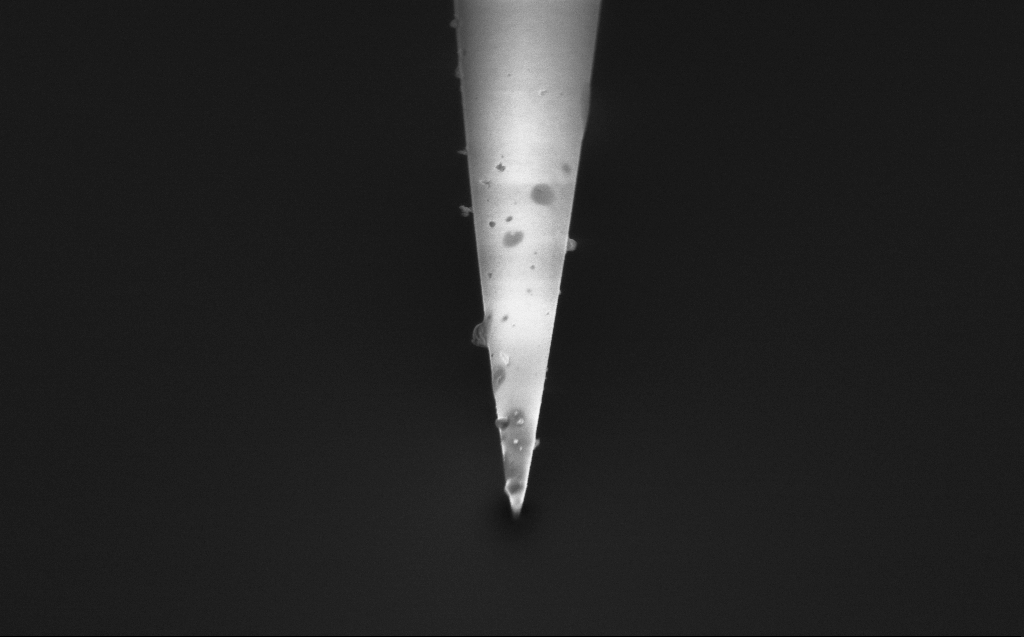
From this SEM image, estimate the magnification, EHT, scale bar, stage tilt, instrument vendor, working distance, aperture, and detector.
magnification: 25.57 K X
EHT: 1.2 kV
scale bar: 2000 nm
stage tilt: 45.1°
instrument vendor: Zeiss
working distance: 2 mm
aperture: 20 µm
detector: InLens